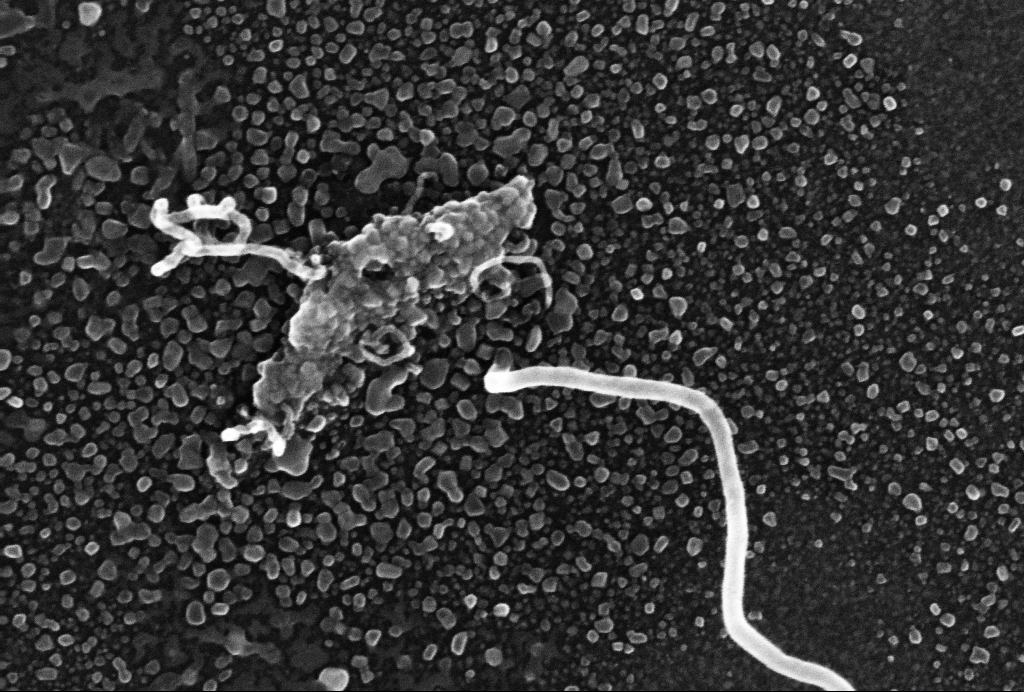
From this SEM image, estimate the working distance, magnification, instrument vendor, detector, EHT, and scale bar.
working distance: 3 mm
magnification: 190.67 K X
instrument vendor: Zeiss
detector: InLens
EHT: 10 kV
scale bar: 300 nm